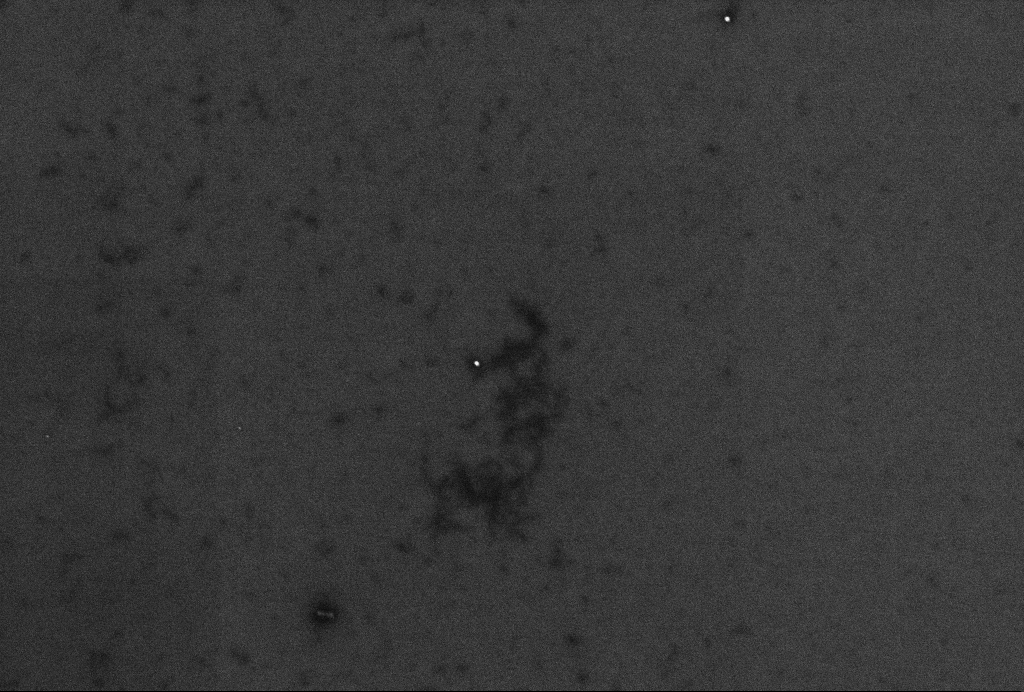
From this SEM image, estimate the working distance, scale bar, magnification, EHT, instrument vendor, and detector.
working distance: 3.3 mm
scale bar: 200 nm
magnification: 62.65 K X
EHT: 2 kV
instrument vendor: Zeiss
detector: InLens